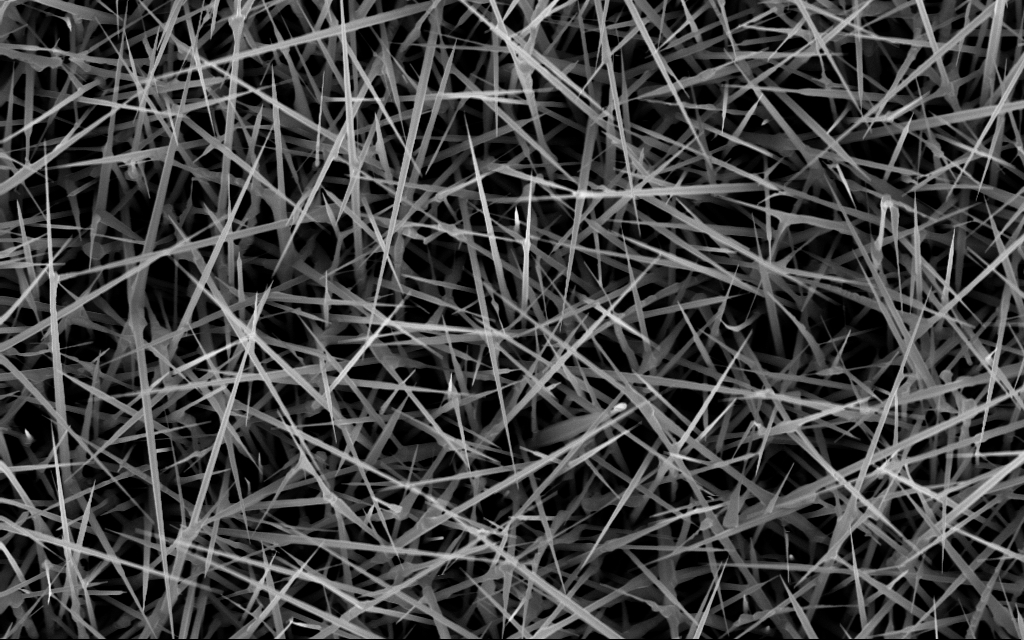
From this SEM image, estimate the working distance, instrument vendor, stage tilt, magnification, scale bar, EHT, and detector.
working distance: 7 mm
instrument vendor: Zeiss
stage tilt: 0°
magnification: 20 K X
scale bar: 2000 nm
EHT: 10 kV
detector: InLens